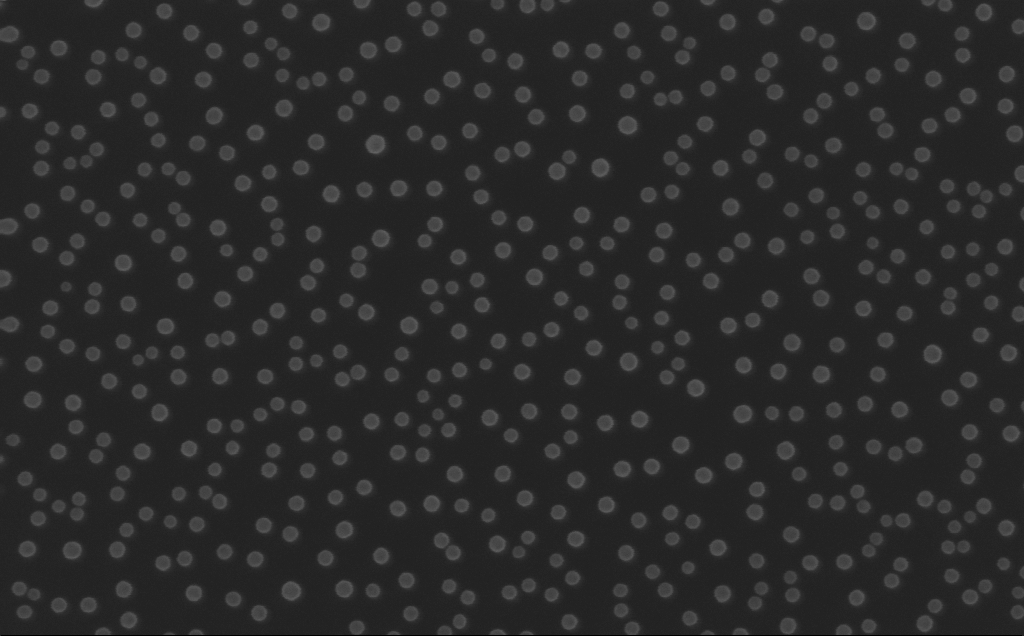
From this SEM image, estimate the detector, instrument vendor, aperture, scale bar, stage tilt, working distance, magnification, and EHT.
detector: InLens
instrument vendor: Zeiss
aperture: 30 µm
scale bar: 100 nm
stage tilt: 0°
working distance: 4 mm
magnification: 200 K X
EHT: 10 kV